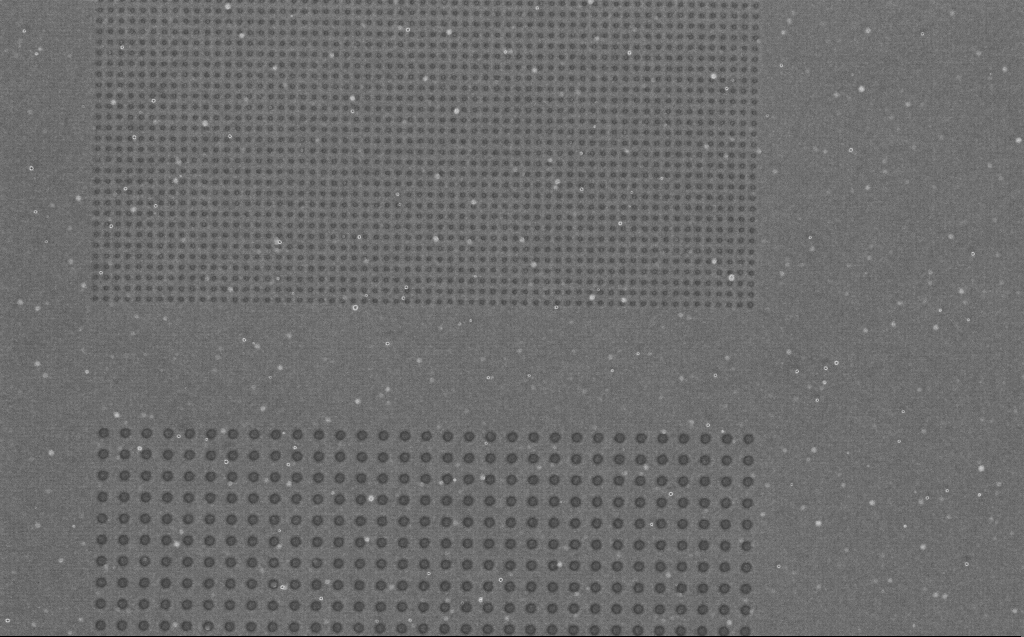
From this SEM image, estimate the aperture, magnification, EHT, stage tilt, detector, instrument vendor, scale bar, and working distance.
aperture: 30 µm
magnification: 9.92 K X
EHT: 1 kV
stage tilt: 0°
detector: InLens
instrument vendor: Zeiss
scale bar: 2000 nm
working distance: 4 mm